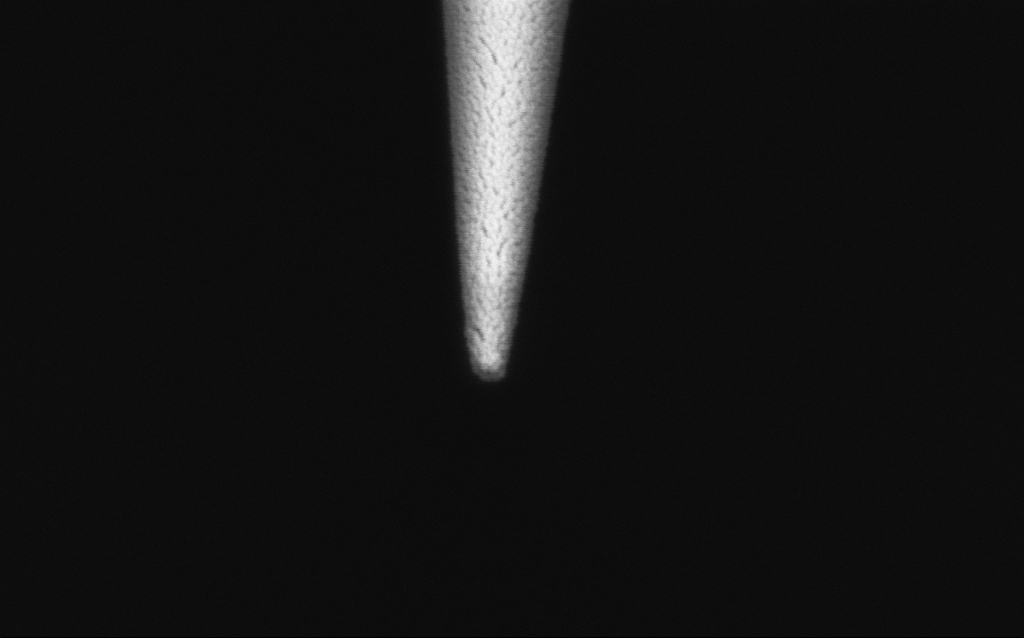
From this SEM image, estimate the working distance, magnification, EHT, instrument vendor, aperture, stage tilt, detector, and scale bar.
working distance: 7.8 mm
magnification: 150 K X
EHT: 2 kV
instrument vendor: Zeiss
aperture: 30 µm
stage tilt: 45°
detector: InLens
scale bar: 200 nm